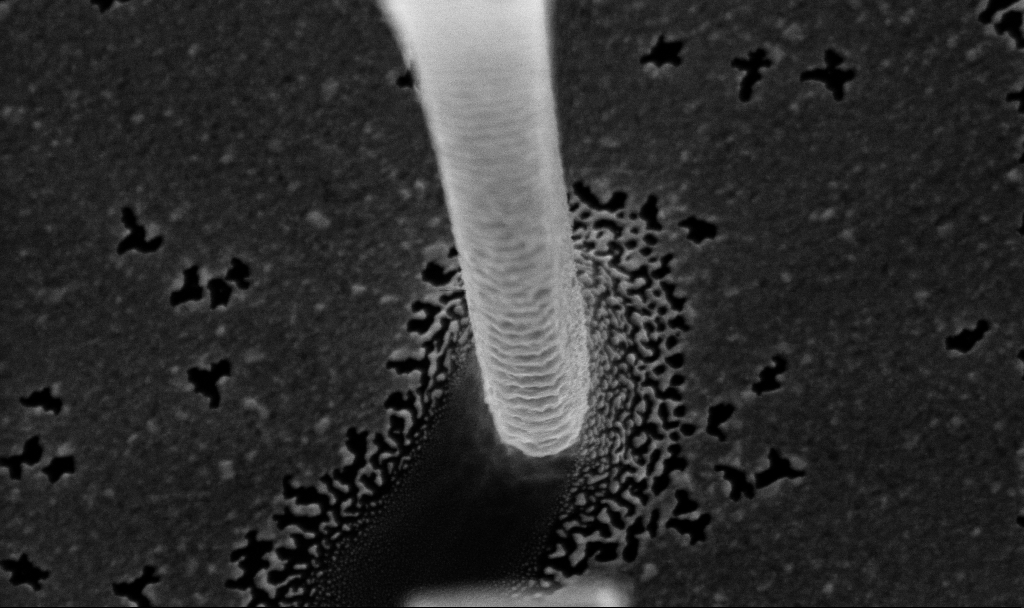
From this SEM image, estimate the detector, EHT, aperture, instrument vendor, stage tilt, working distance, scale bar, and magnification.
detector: InLens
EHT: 5 kV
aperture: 30 µm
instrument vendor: Zeiss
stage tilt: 12°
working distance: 4.6 mm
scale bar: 1000 nm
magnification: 54.84 K X